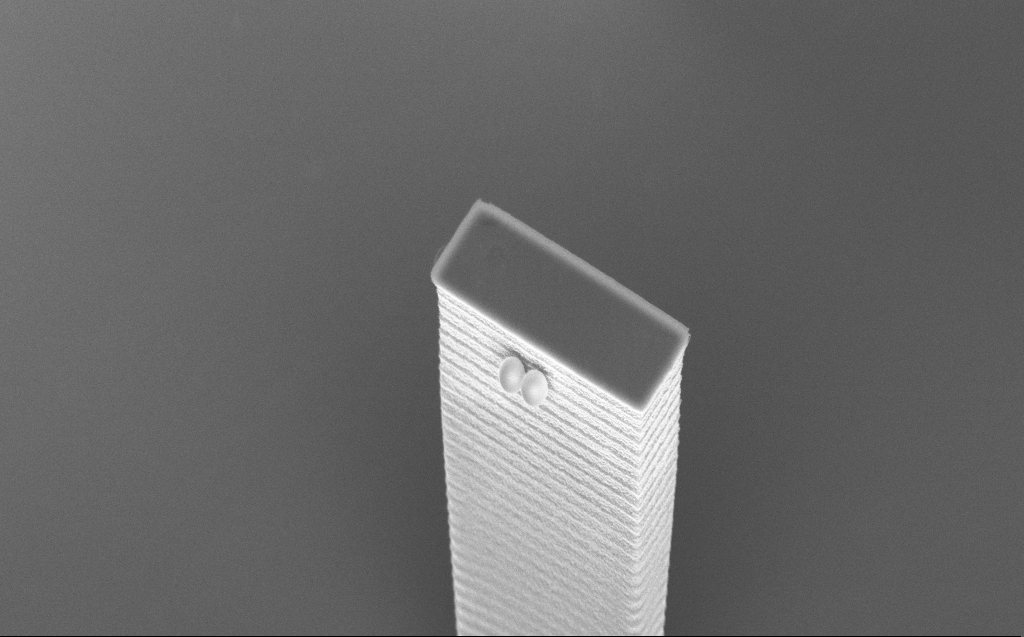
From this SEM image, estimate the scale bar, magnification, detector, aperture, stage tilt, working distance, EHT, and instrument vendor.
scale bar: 1000 nm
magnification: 12.31 K X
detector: InLens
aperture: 30 µm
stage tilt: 45°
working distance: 7 mm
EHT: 5 kV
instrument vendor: Zeiss